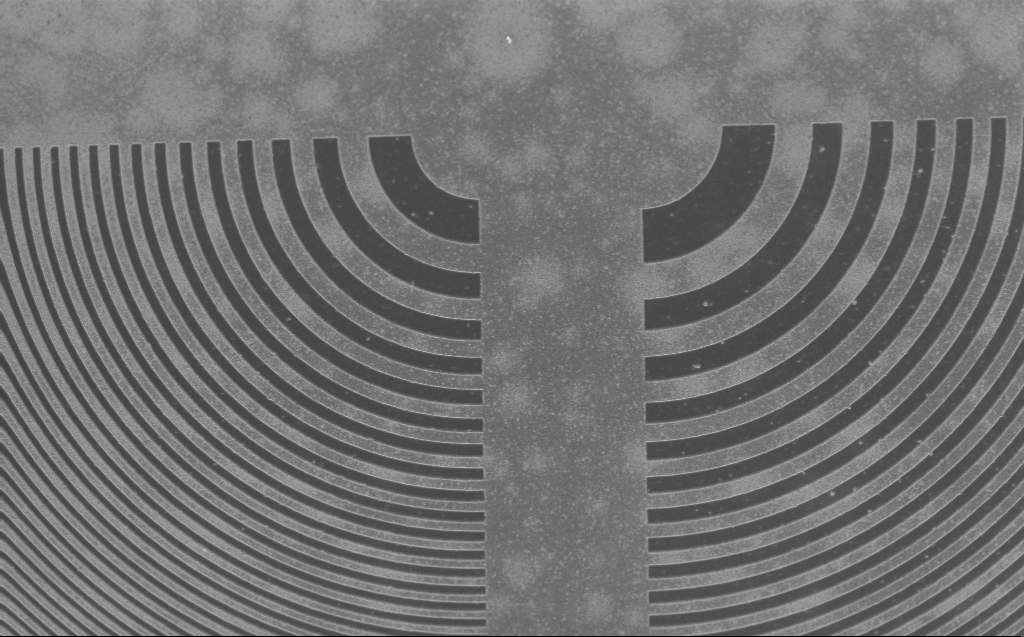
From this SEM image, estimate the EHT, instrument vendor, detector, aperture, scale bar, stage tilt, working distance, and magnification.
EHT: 2.5 kV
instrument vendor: Zeiss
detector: InLens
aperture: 30 µm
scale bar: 10000 nm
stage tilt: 30°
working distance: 4 mm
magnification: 5.98 K X